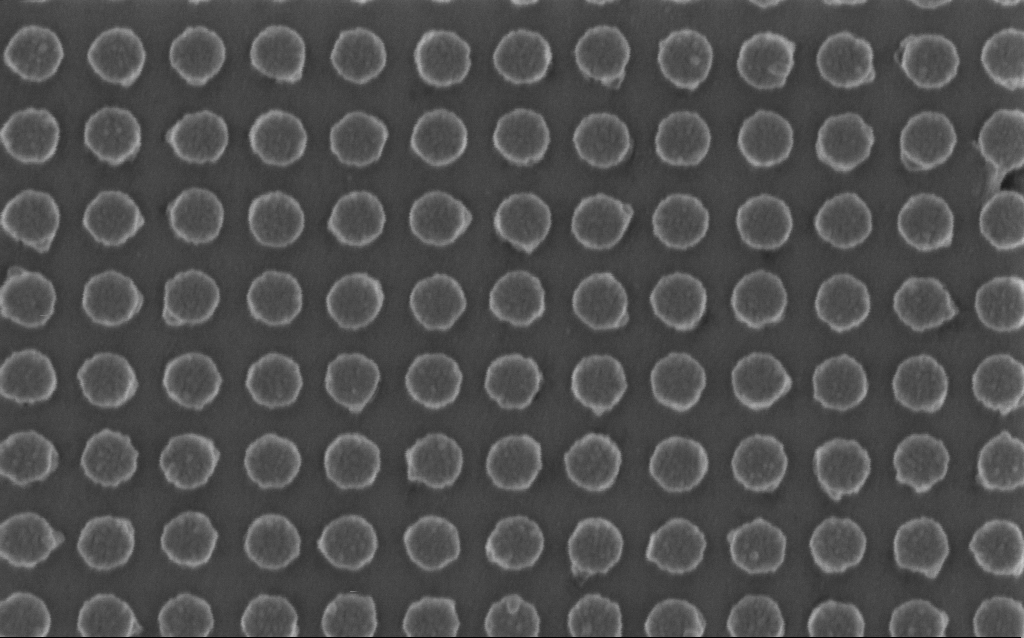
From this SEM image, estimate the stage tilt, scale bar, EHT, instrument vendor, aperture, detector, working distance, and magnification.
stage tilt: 0°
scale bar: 200 nm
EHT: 1.5 kV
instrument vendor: Zeiss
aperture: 30 µm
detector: InLens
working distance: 6 mm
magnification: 98.63 K X